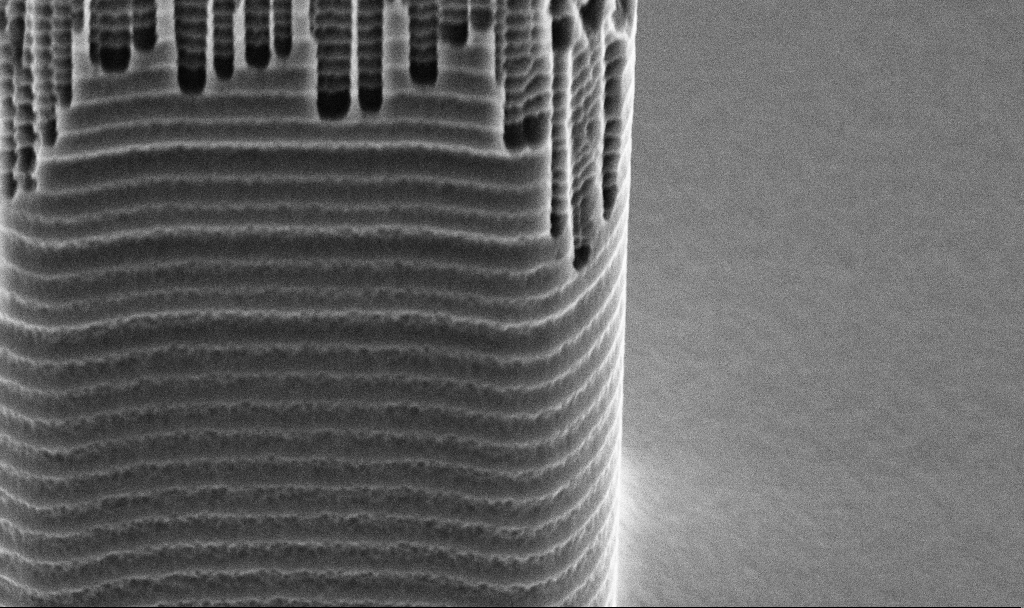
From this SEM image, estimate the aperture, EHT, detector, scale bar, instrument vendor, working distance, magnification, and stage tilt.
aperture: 30 µm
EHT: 5 kV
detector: SE2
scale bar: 2000 nm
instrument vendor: Zeiss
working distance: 11.6 mm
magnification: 24.51 K X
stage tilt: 45°